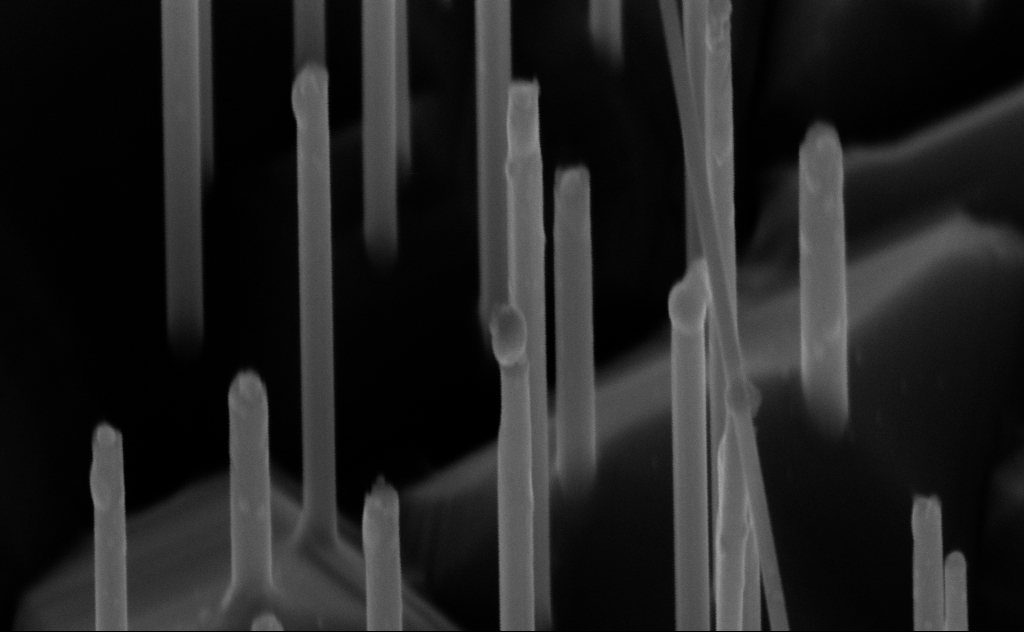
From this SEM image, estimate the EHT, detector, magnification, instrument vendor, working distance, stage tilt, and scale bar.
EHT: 10 kV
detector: InLens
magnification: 180.63 K X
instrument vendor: Zeiss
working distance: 6 mm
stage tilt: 45°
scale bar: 200 nm